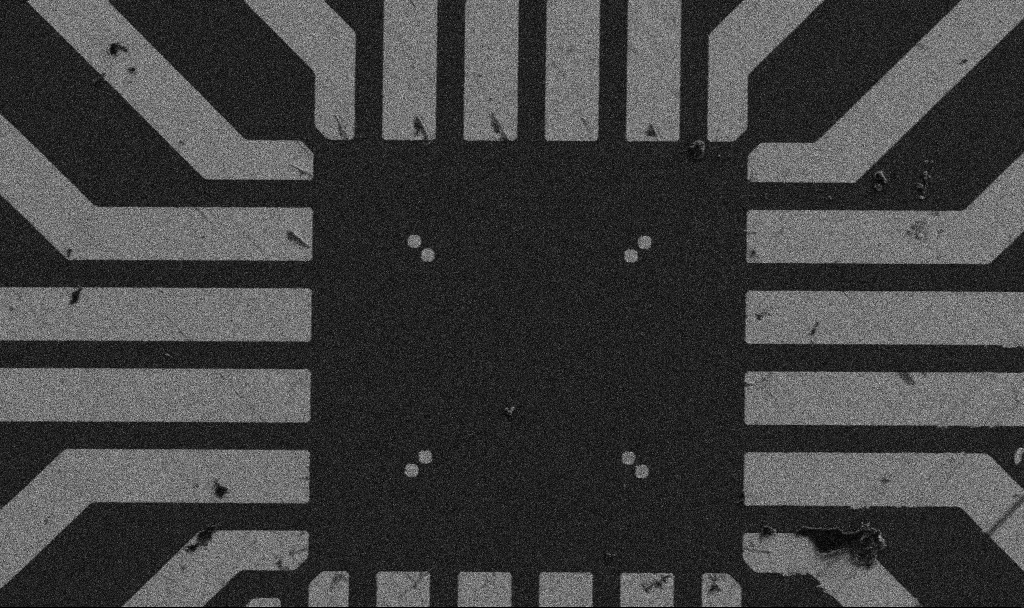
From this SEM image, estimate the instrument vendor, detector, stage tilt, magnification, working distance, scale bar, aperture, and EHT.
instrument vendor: Zeiss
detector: SE2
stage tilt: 0°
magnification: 1 K X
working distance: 8.9 mm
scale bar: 20000 nm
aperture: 30 µm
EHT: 5 kV